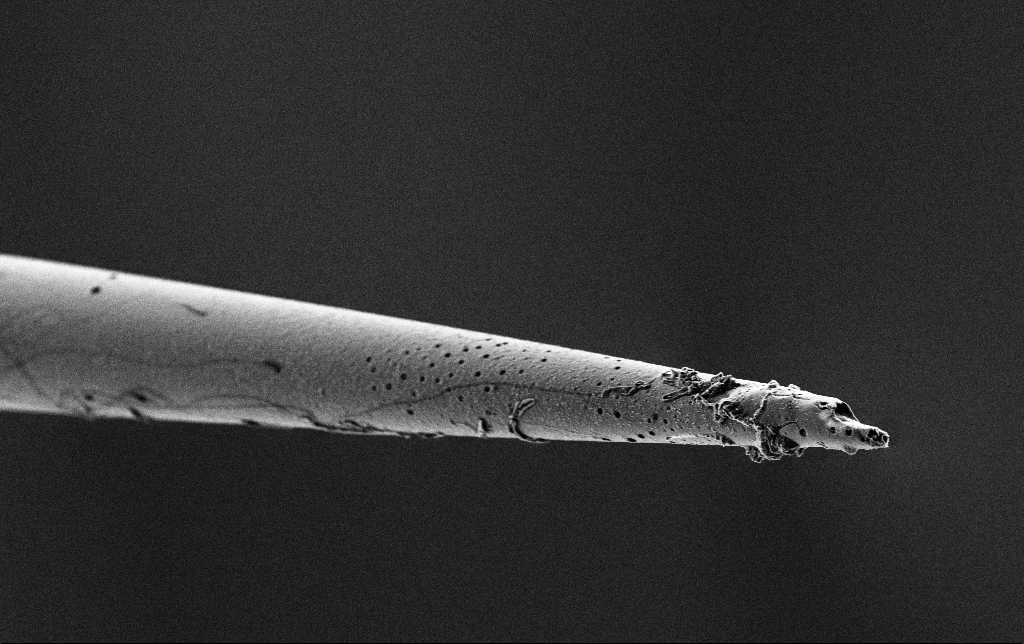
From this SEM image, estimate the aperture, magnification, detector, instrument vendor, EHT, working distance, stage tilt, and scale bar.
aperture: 30 µm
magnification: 5 K X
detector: SE2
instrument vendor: Zeiss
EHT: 2 kV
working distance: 7.2 mm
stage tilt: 45°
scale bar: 10000 nm